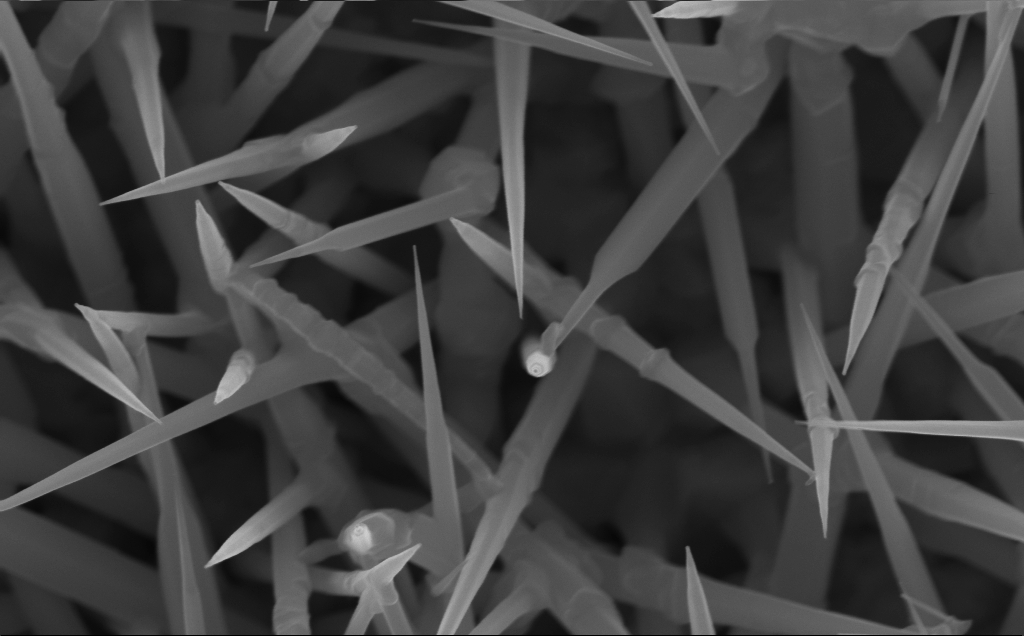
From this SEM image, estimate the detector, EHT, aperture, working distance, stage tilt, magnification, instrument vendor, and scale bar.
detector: InLens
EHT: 10 kV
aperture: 30 µm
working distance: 4 mm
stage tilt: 0°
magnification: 80 K X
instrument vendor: Zeiss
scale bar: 200 nm